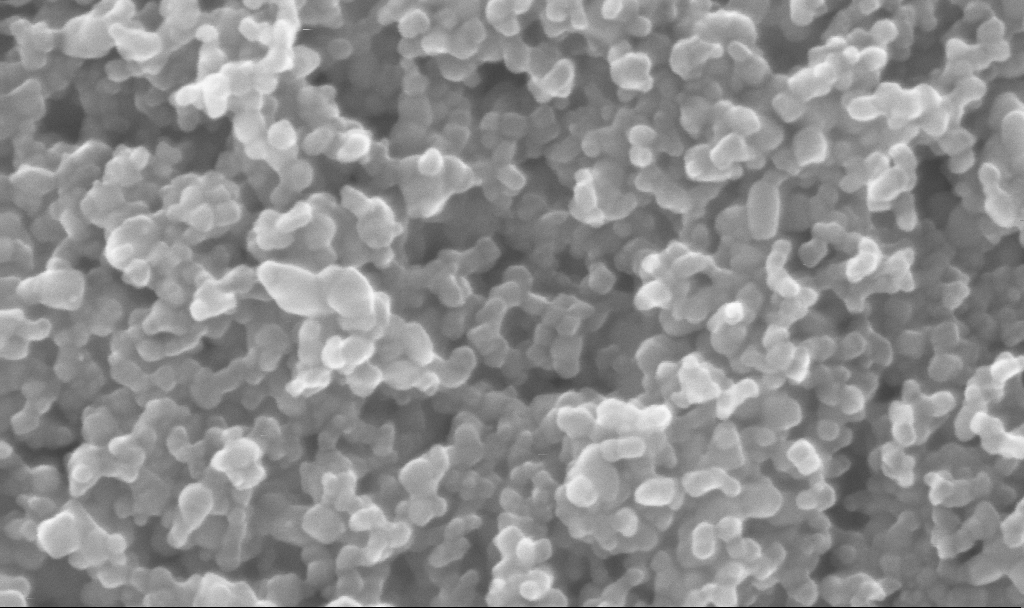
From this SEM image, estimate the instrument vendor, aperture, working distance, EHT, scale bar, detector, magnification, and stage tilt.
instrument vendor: Zeiss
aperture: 30 µm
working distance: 2.7 mm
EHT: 10 kV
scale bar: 200 nm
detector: InLens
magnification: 325.39 K X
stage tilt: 0°